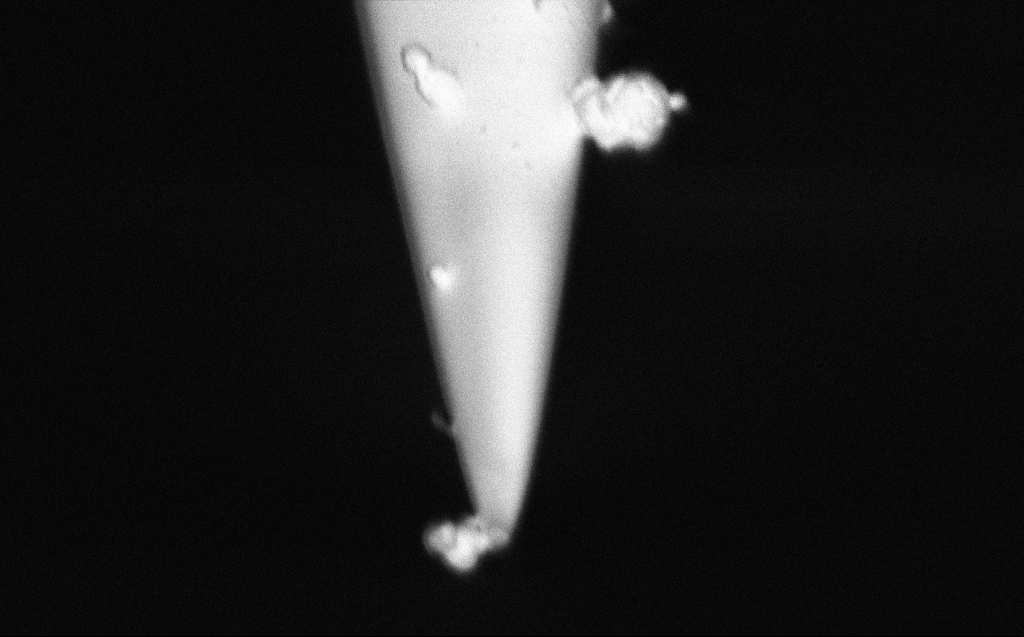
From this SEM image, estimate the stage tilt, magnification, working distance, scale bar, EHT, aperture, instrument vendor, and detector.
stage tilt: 45.1°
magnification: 86.07 K X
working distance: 5 mm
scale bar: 200 nm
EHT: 1 kV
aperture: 30 µm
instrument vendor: Zeiss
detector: InLens